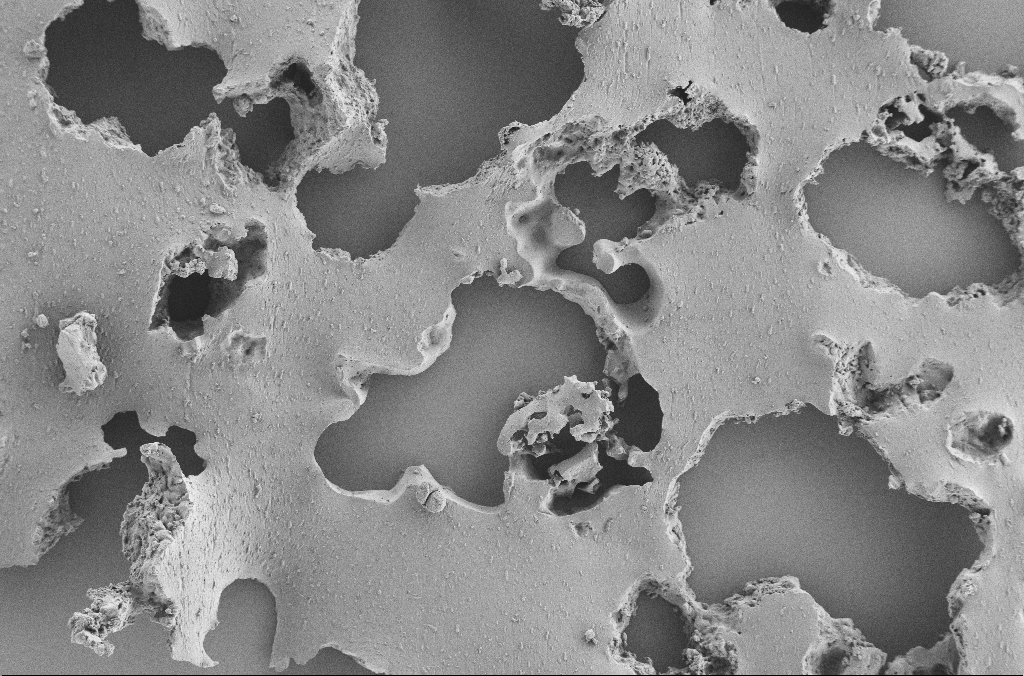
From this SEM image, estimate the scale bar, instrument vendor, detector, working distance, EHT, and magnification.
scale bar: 100000 nm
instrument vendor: Zeiss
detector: SE2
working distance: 3.6 mm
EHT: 2 kV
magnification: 0.25 K X